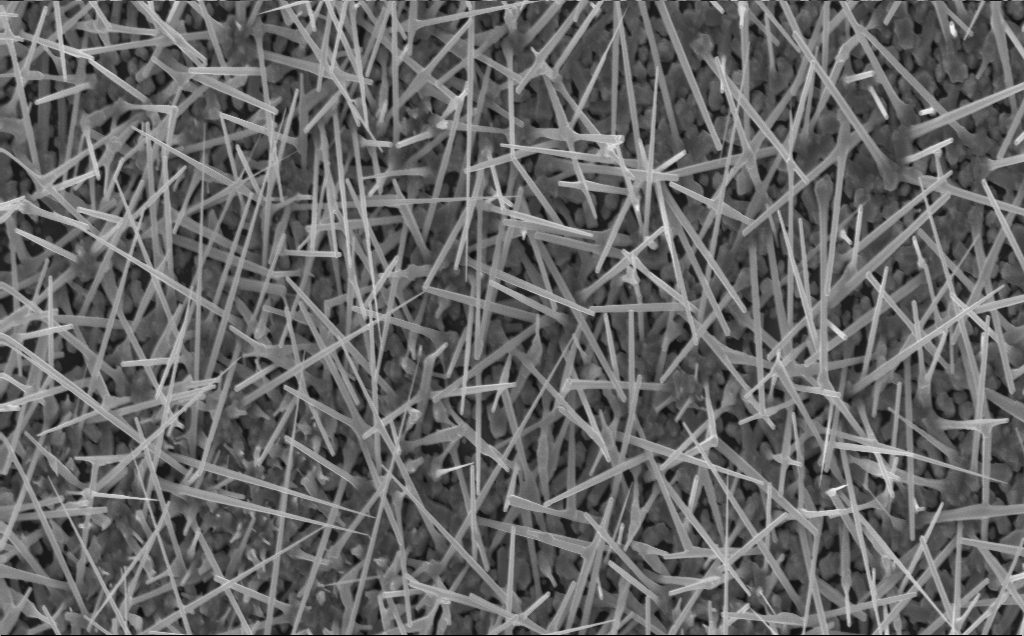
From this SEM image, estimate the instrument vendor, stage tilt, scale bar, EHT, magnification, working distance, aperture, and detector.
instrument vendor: Zeiss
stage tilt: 0°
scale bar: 2000 nm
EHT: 10 kV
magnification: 10 K X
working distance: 4 mm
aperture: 30 µm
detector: InLens